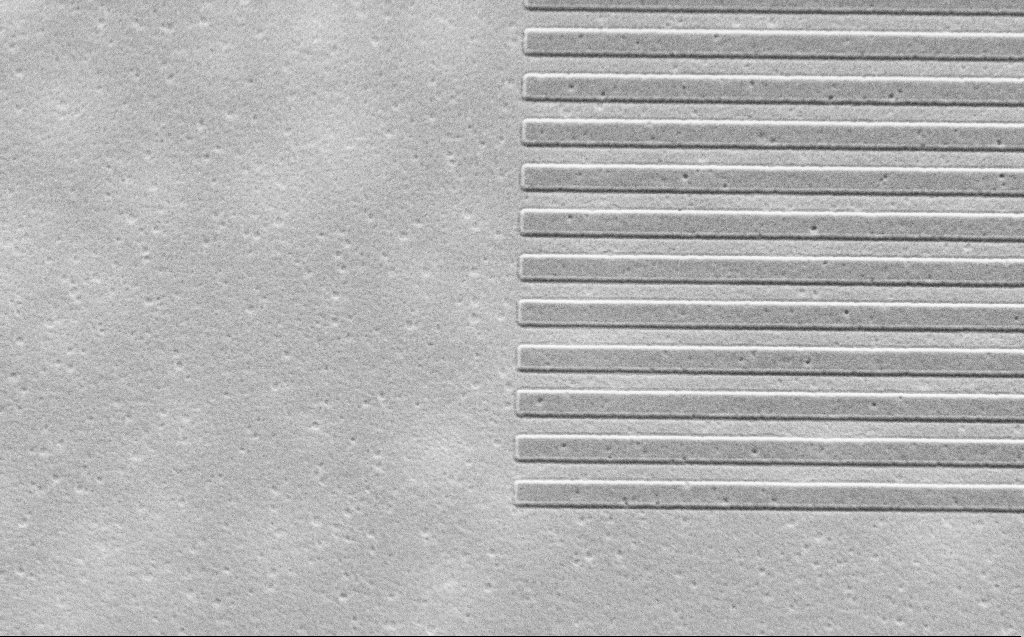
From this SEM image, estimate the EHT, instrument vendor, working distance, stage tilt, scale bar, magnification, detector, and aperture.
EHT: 2.5 kV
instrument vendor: Zeiss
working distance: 4 mm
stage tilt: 30°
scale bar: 2000 nm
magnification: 14.1 K X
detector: SE2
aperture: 30 µm